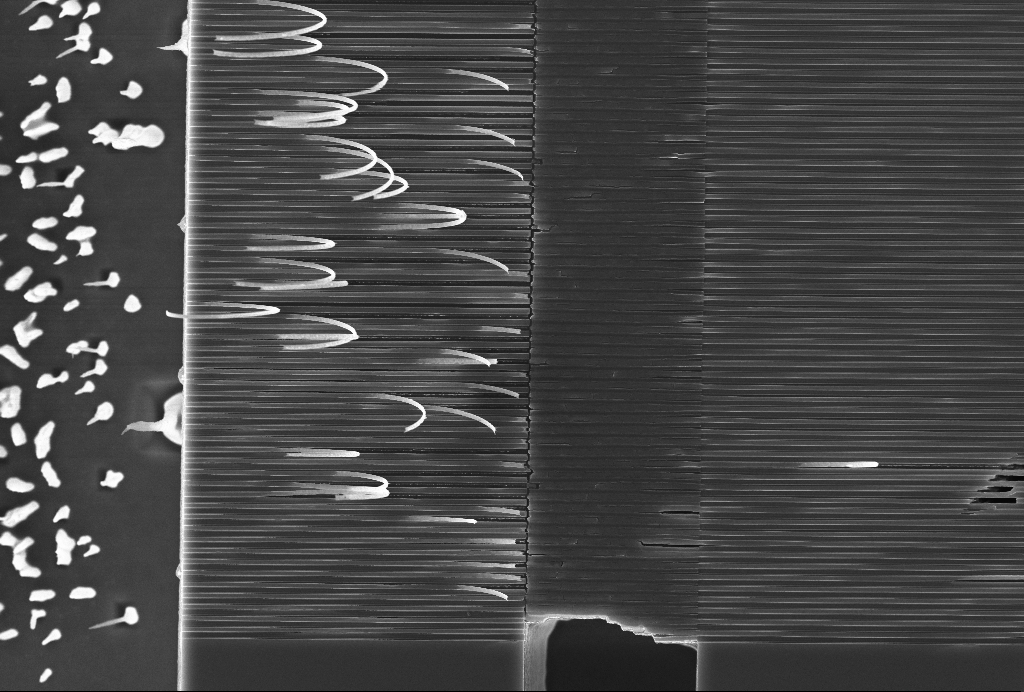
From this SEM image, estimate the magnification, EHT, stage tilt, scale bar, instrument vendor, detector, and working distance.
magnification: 6.3 K X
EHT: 10 kV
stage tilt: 0°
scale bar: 2000 nm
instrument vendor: Zeiss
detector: InLens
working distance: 5 mm